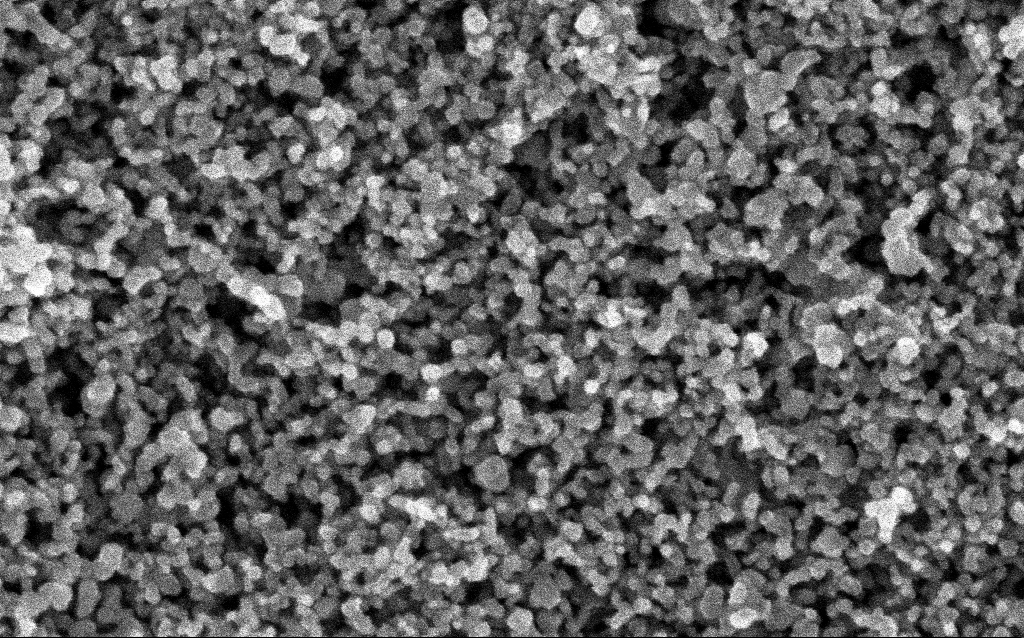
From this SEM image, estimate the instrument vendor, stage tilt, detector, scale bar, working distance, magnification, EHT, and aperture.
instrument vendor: Zeiss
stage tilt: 0°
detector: InLens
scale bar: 100 nm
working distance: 4.9 mm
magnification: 204.13 K X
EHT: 5 kV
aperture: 30 µm